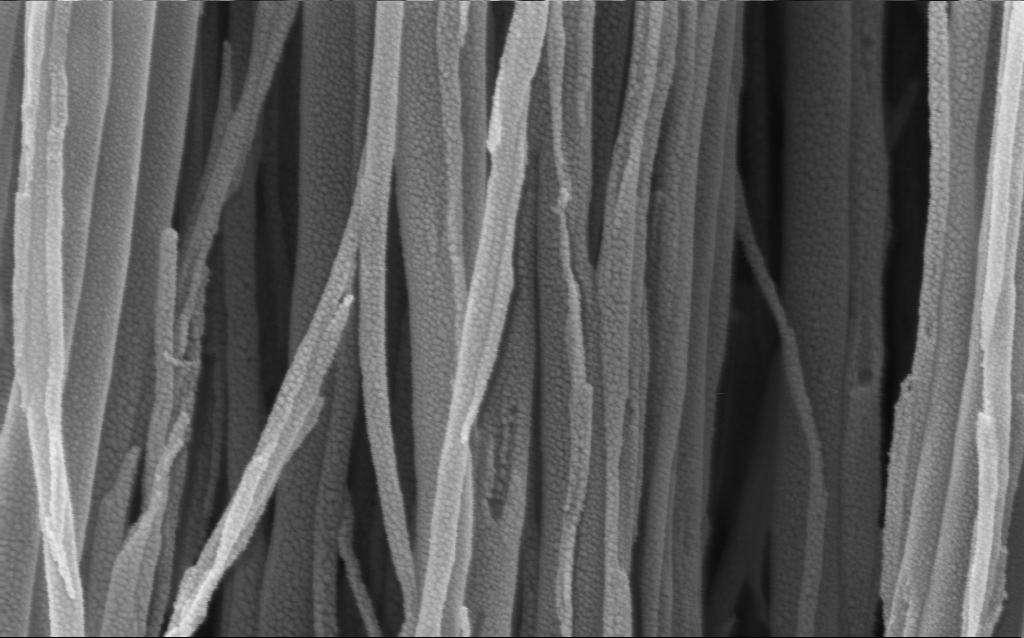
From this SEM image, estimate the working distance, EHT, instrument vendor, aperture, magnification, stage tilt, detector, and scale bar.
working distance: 3 mm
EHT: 3 kV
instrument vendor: Zeiss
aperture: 30 µm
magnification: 135.4 K X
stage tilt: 0°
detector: InLens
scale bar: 200 nm